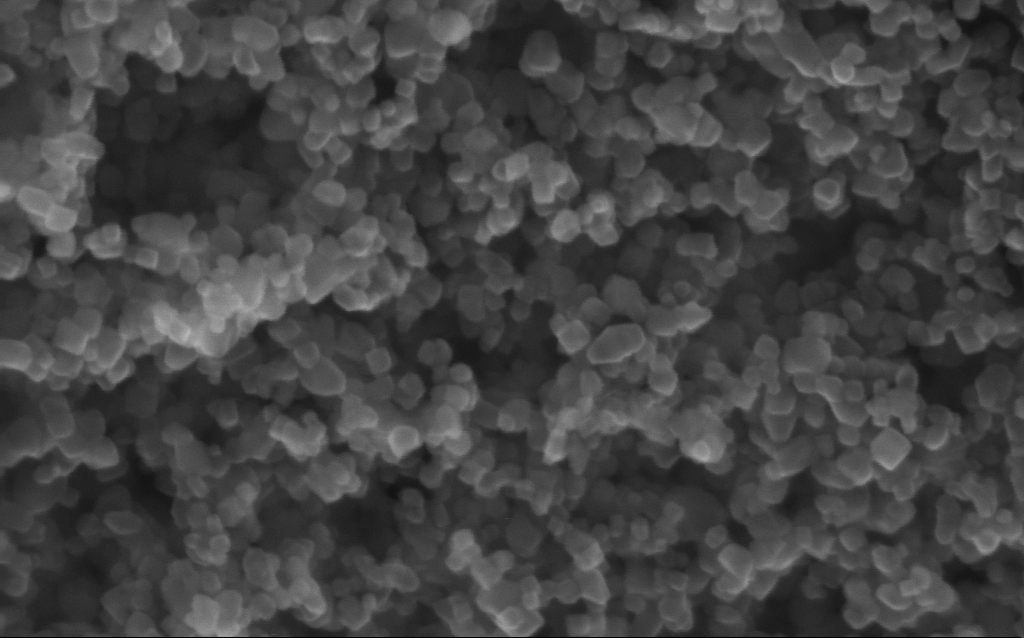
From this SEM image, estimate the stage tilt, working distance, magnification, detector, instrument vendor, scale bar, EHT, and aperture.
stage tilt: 0°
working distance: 2.7 mm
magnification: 416 K X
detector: InLens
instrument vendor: Zeiss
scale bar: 100 nm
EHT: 10 kV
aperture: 30 µm